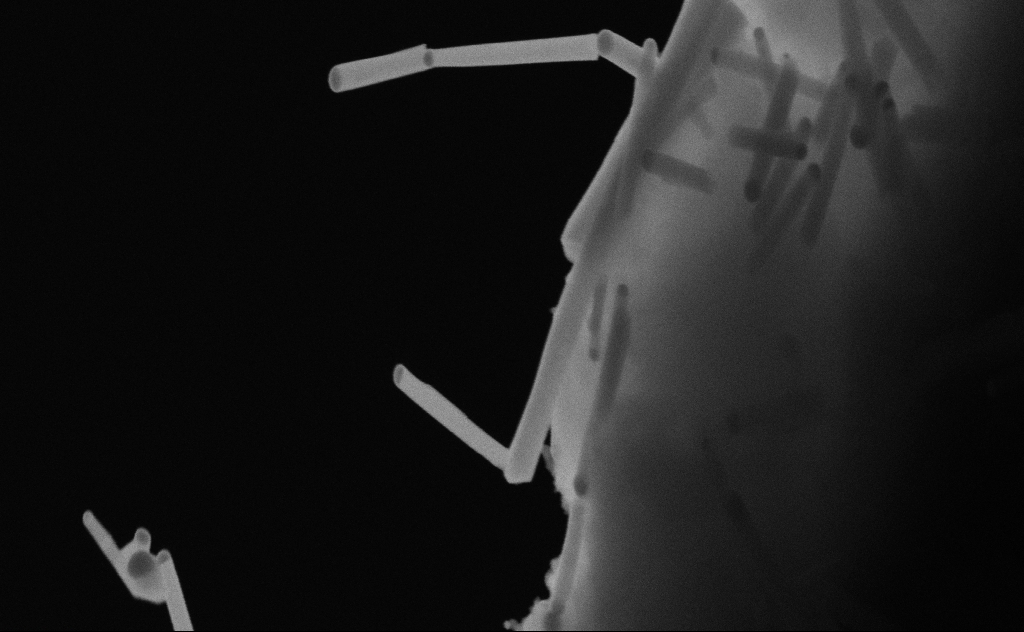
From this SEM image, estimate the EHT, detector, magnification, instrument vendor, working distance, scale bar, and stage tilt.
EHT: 20 kV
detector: SE2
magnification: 103.29 K X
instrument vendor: Zeiss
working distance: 9 mm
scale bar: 200 nm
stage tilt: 0°